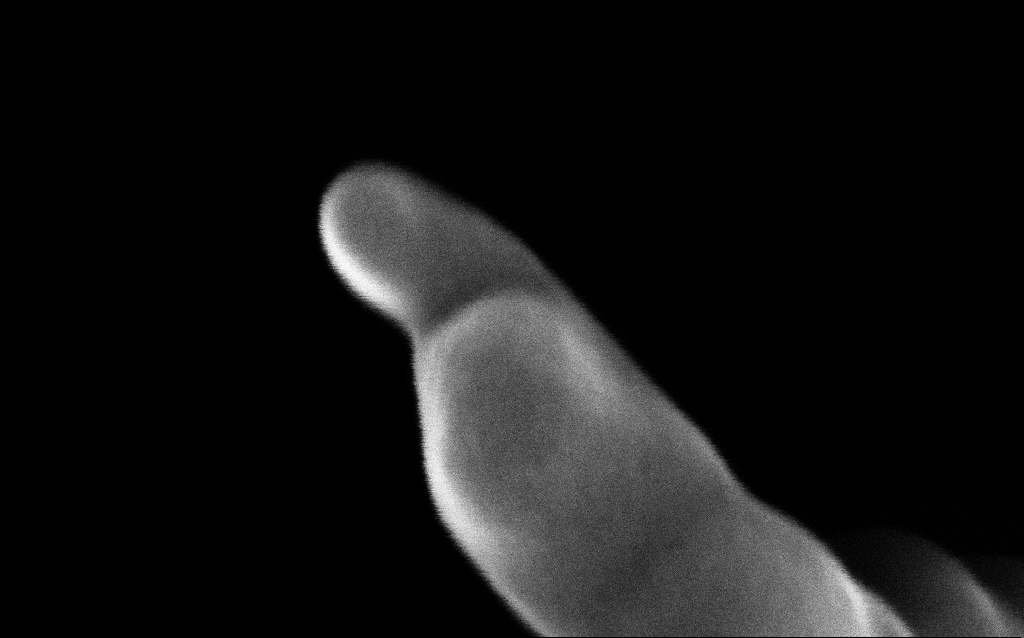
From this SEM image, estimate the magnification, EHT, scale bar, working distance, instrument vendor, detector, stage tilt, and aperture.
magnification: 747.82 K X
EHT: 10 kV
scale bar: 20 nm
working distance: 3.7 mm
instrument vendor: Zeiss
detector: InLens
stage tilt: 45°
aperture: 30 µm